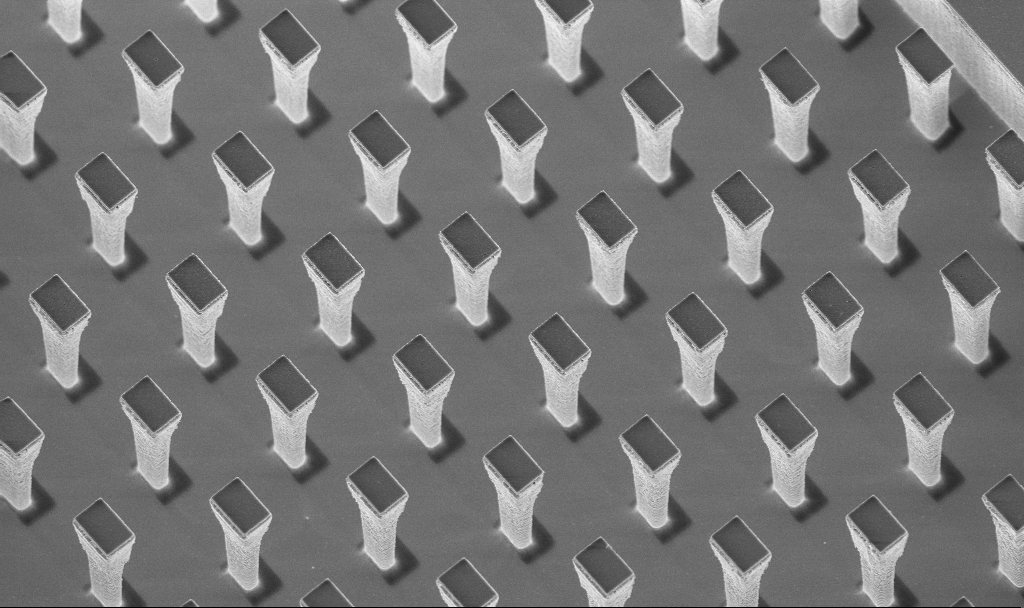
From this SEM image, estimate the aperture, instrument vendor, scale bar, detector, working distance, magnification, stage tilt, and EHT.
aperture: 30 µm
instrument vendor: Zeiss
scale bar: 10000 nm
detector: InLens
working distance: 4.3 mm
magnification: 4.34 K X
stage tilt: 20°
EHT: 5 kV